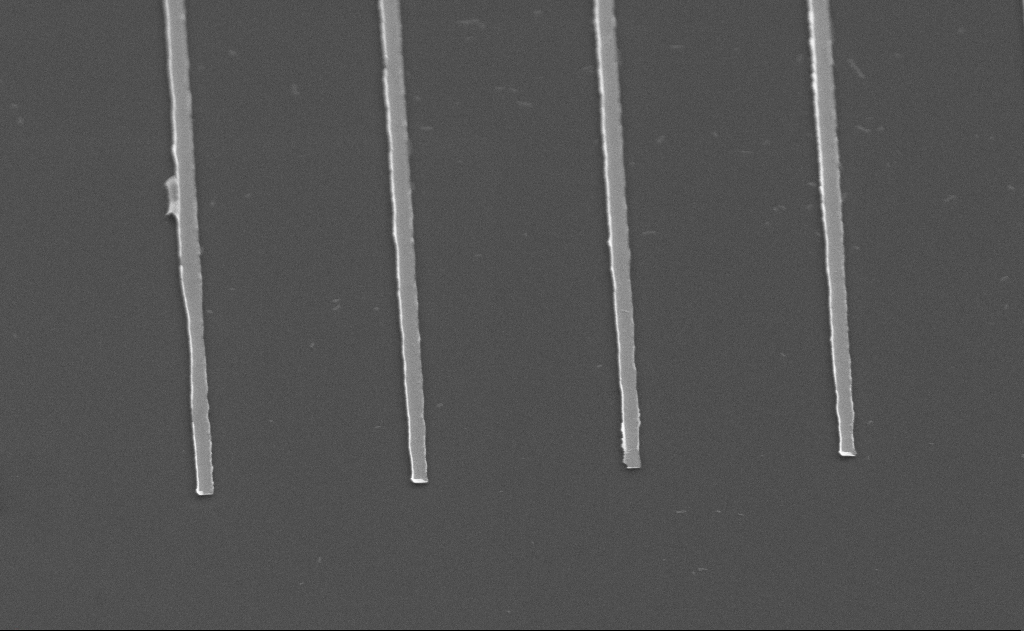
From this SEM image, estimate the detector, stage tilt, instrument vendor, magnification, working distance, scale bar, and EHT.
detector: SE2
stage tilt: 45°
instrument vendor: Zeiss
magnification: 19.52 K X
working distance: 12 mm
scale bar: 2000 nm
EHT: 5 kV